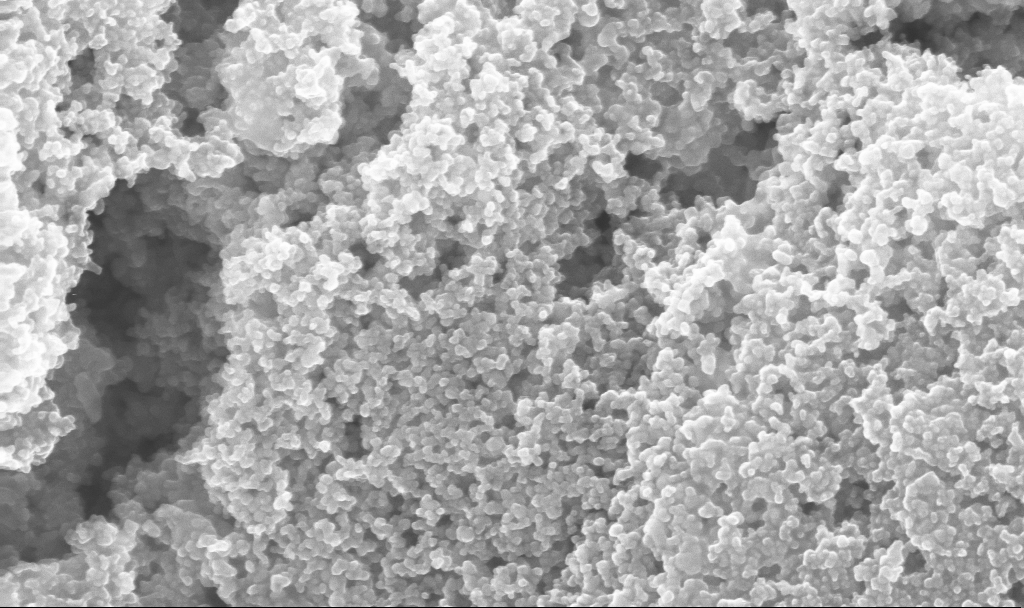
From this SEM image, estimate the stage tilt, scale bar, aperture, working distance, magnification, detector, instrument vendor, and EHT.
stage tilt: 0°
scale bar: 200 nm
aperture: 30 µm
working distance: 2.7 mm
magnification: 101.55 K X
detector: InLens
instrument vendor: Zeiss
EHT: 10 kV